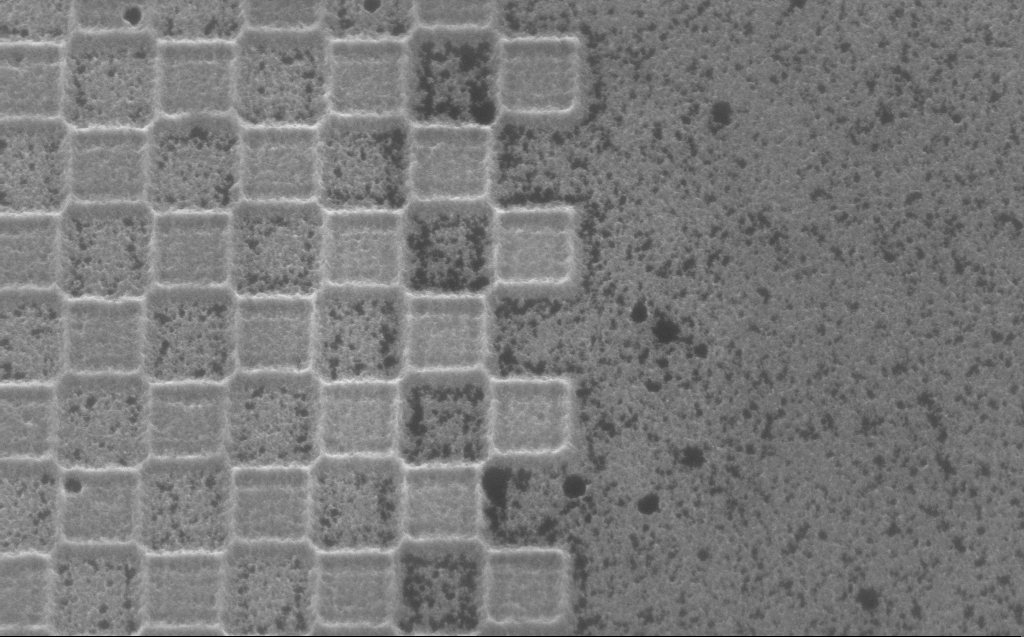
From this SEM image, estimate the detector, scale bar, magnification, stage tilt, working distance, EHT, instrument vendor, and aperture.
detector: InLens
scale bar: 1000 nm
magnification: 63.65 K X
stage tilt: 30°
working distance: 4 mm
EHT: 5 kV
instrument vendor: Zeiss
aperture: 30 µm